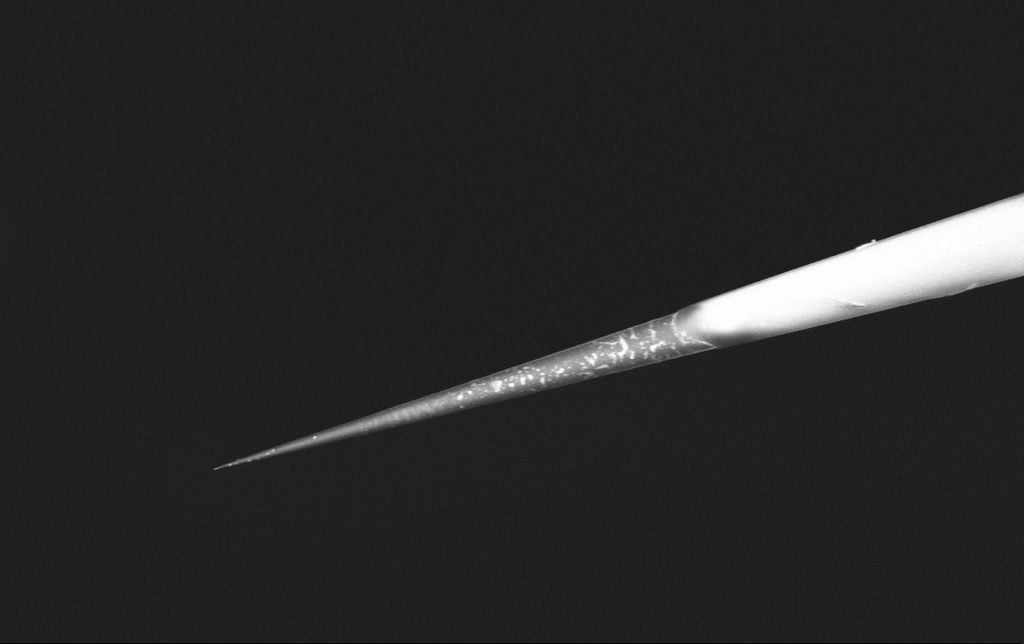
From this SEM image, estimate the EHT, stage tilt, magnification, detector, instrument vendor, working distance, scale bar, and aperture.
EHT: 3 kV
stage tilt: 0°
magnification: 3.5 K X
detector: InLens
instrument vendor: Zeiss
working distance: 6.3 mm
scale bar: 10000 nm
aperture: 30 µm